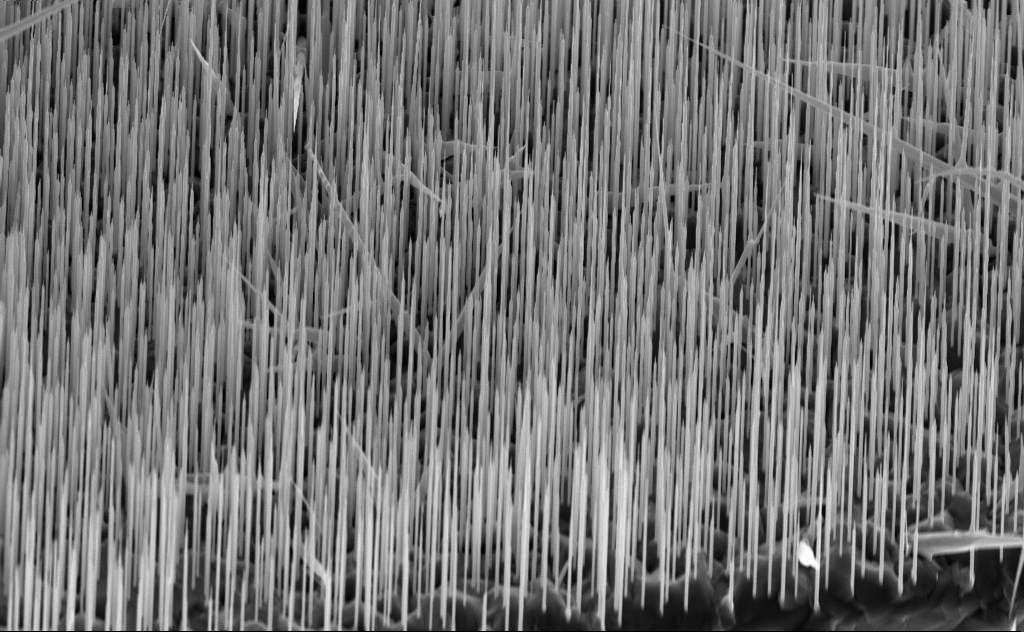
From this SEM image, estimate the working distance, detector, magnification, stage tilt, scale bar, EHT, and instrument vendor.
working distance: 7 mm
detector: InLens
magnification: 10.92 K X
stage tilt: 45°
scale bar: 2000 nm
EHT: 10 kV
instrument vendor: Zeiss